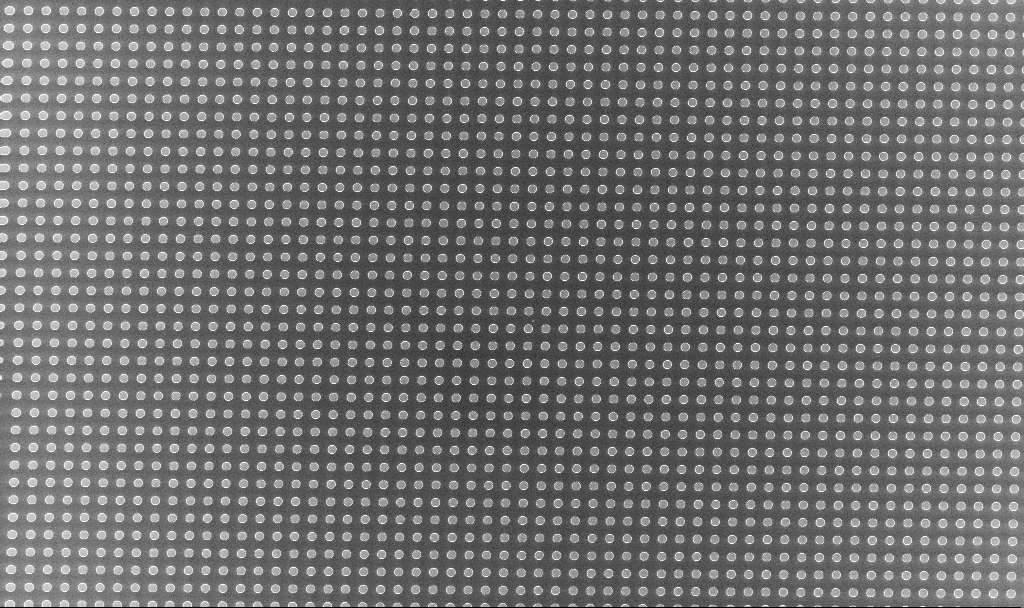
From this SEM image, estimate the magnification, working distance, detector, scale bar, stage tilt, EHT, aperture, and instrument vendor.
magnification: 1.08 K X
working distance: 3.2 mm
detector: InLens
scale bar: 20000 nm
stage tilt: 0°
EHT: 3 kV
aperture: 30 µm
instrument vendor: Zeiss